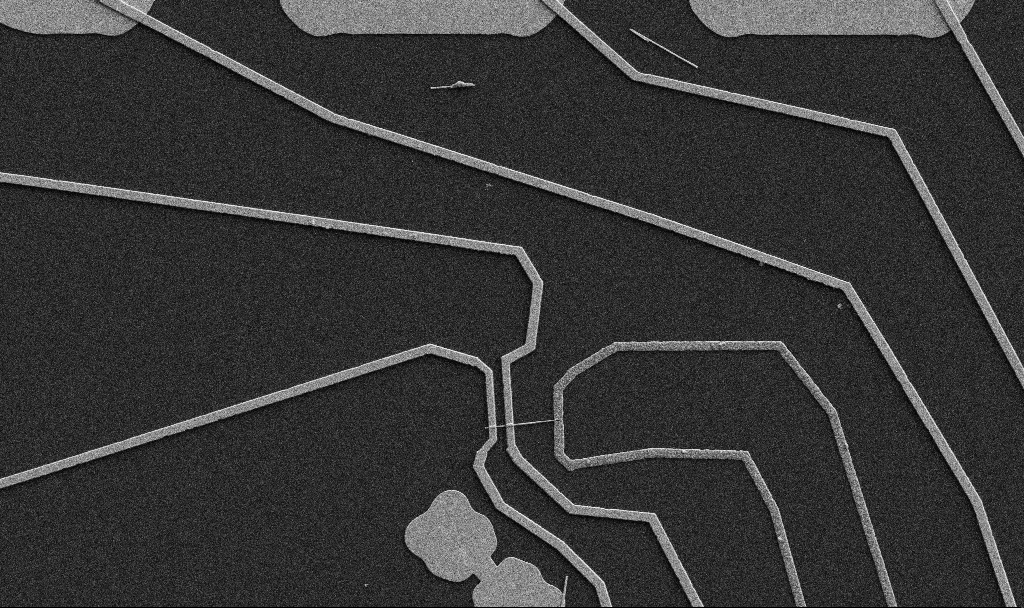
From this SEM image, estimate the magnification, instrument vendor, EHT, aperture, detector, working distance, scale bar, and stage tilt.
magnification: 5 K X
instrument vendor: Zeiss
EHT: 5 kV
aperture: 30 µm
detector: SE2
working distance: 10.7 mm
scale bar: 10000 nm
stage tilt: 0°